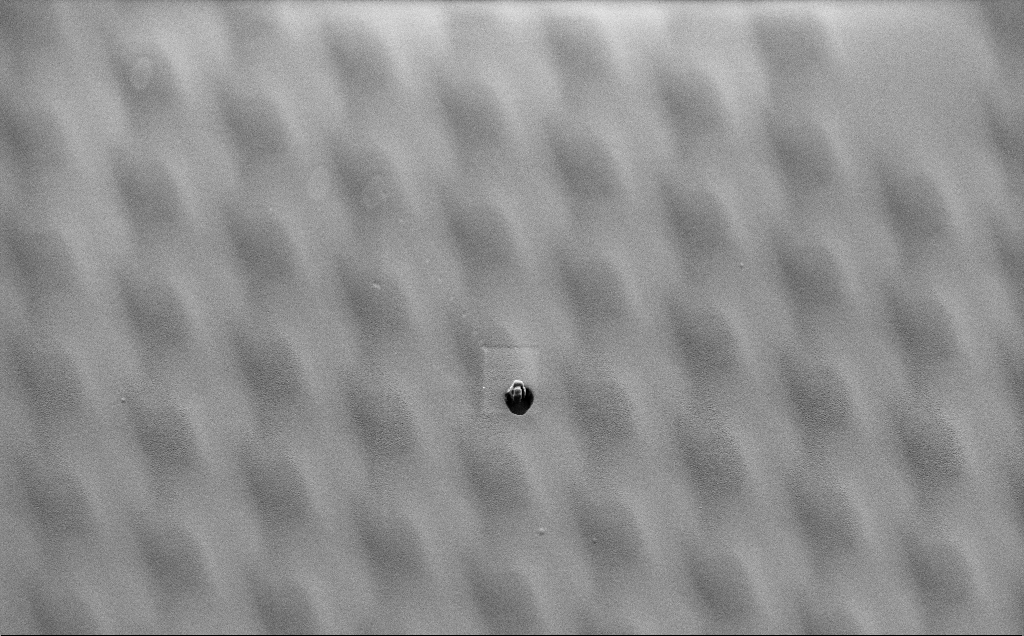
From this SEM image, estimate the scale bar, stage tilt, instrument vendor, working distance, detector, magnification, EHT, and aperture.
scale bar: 10000 nm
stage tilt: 45°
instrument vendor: Zeiss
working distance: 6 mm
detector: SE2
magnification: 4.65 K X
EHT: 1.5 kV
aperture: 30 µm